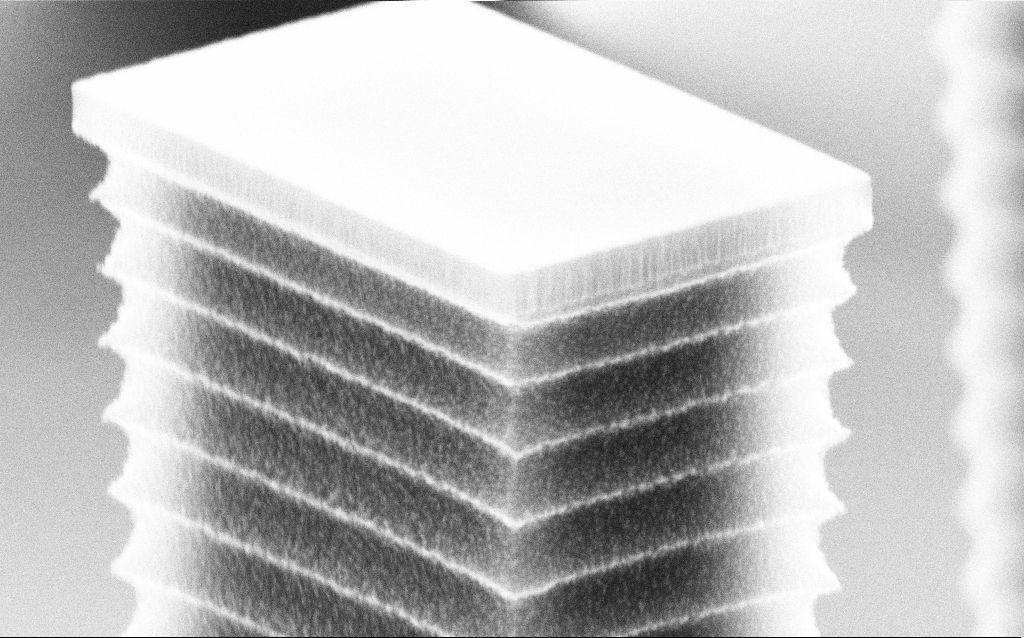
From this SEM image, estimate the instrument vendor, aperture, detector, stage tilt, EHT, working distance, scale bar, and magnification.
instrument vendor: Zeiss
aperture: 30 µm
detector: SE2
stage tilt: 70°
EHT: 8 kV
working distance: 7.5 mm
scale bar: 200 nm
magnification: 86.05 K X